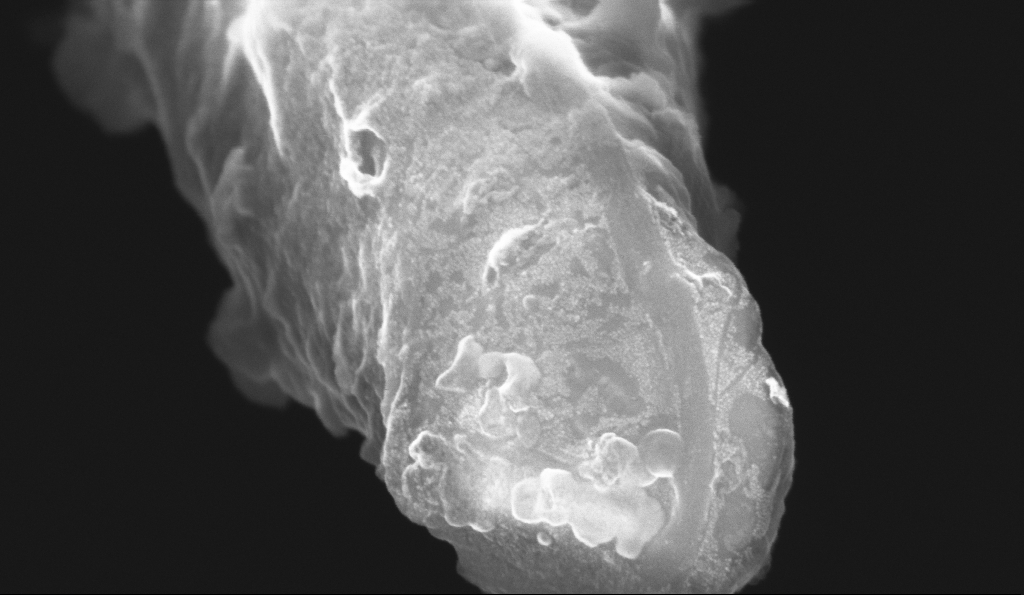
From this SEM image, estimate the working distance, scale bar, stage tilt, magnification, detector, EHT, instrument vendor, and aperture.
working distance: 5.1 mm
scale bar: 100 nm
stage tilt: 70°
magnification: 163.58 K X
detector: InLens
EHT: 10 kV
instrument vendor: Zeiss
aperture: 30 µm